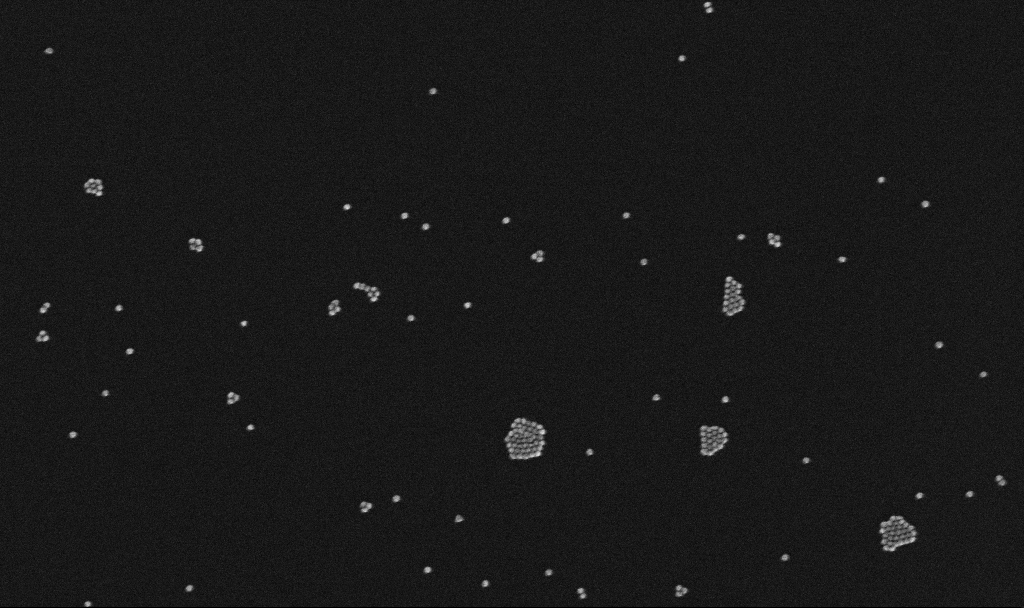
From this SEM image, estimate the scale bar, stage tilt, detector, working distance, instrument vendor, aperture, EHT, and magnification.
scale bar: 200 nm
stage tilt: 0°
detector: InLens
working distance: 3.2 mm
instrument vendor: Zeiss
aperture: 30 µm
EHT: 10 kV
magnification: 100 K X